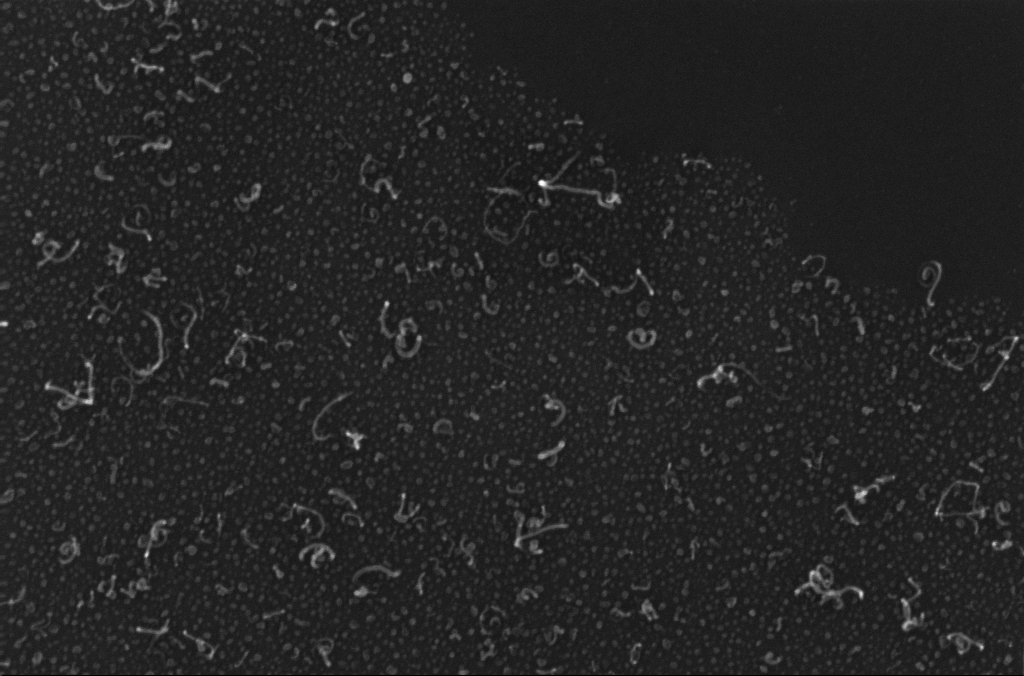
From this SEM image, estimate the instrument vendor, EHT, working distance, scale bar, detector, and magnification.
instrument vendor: Zeiss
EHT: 10 kV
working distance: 3.3 mm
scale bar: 100 nm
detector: InLens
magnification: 150 K X